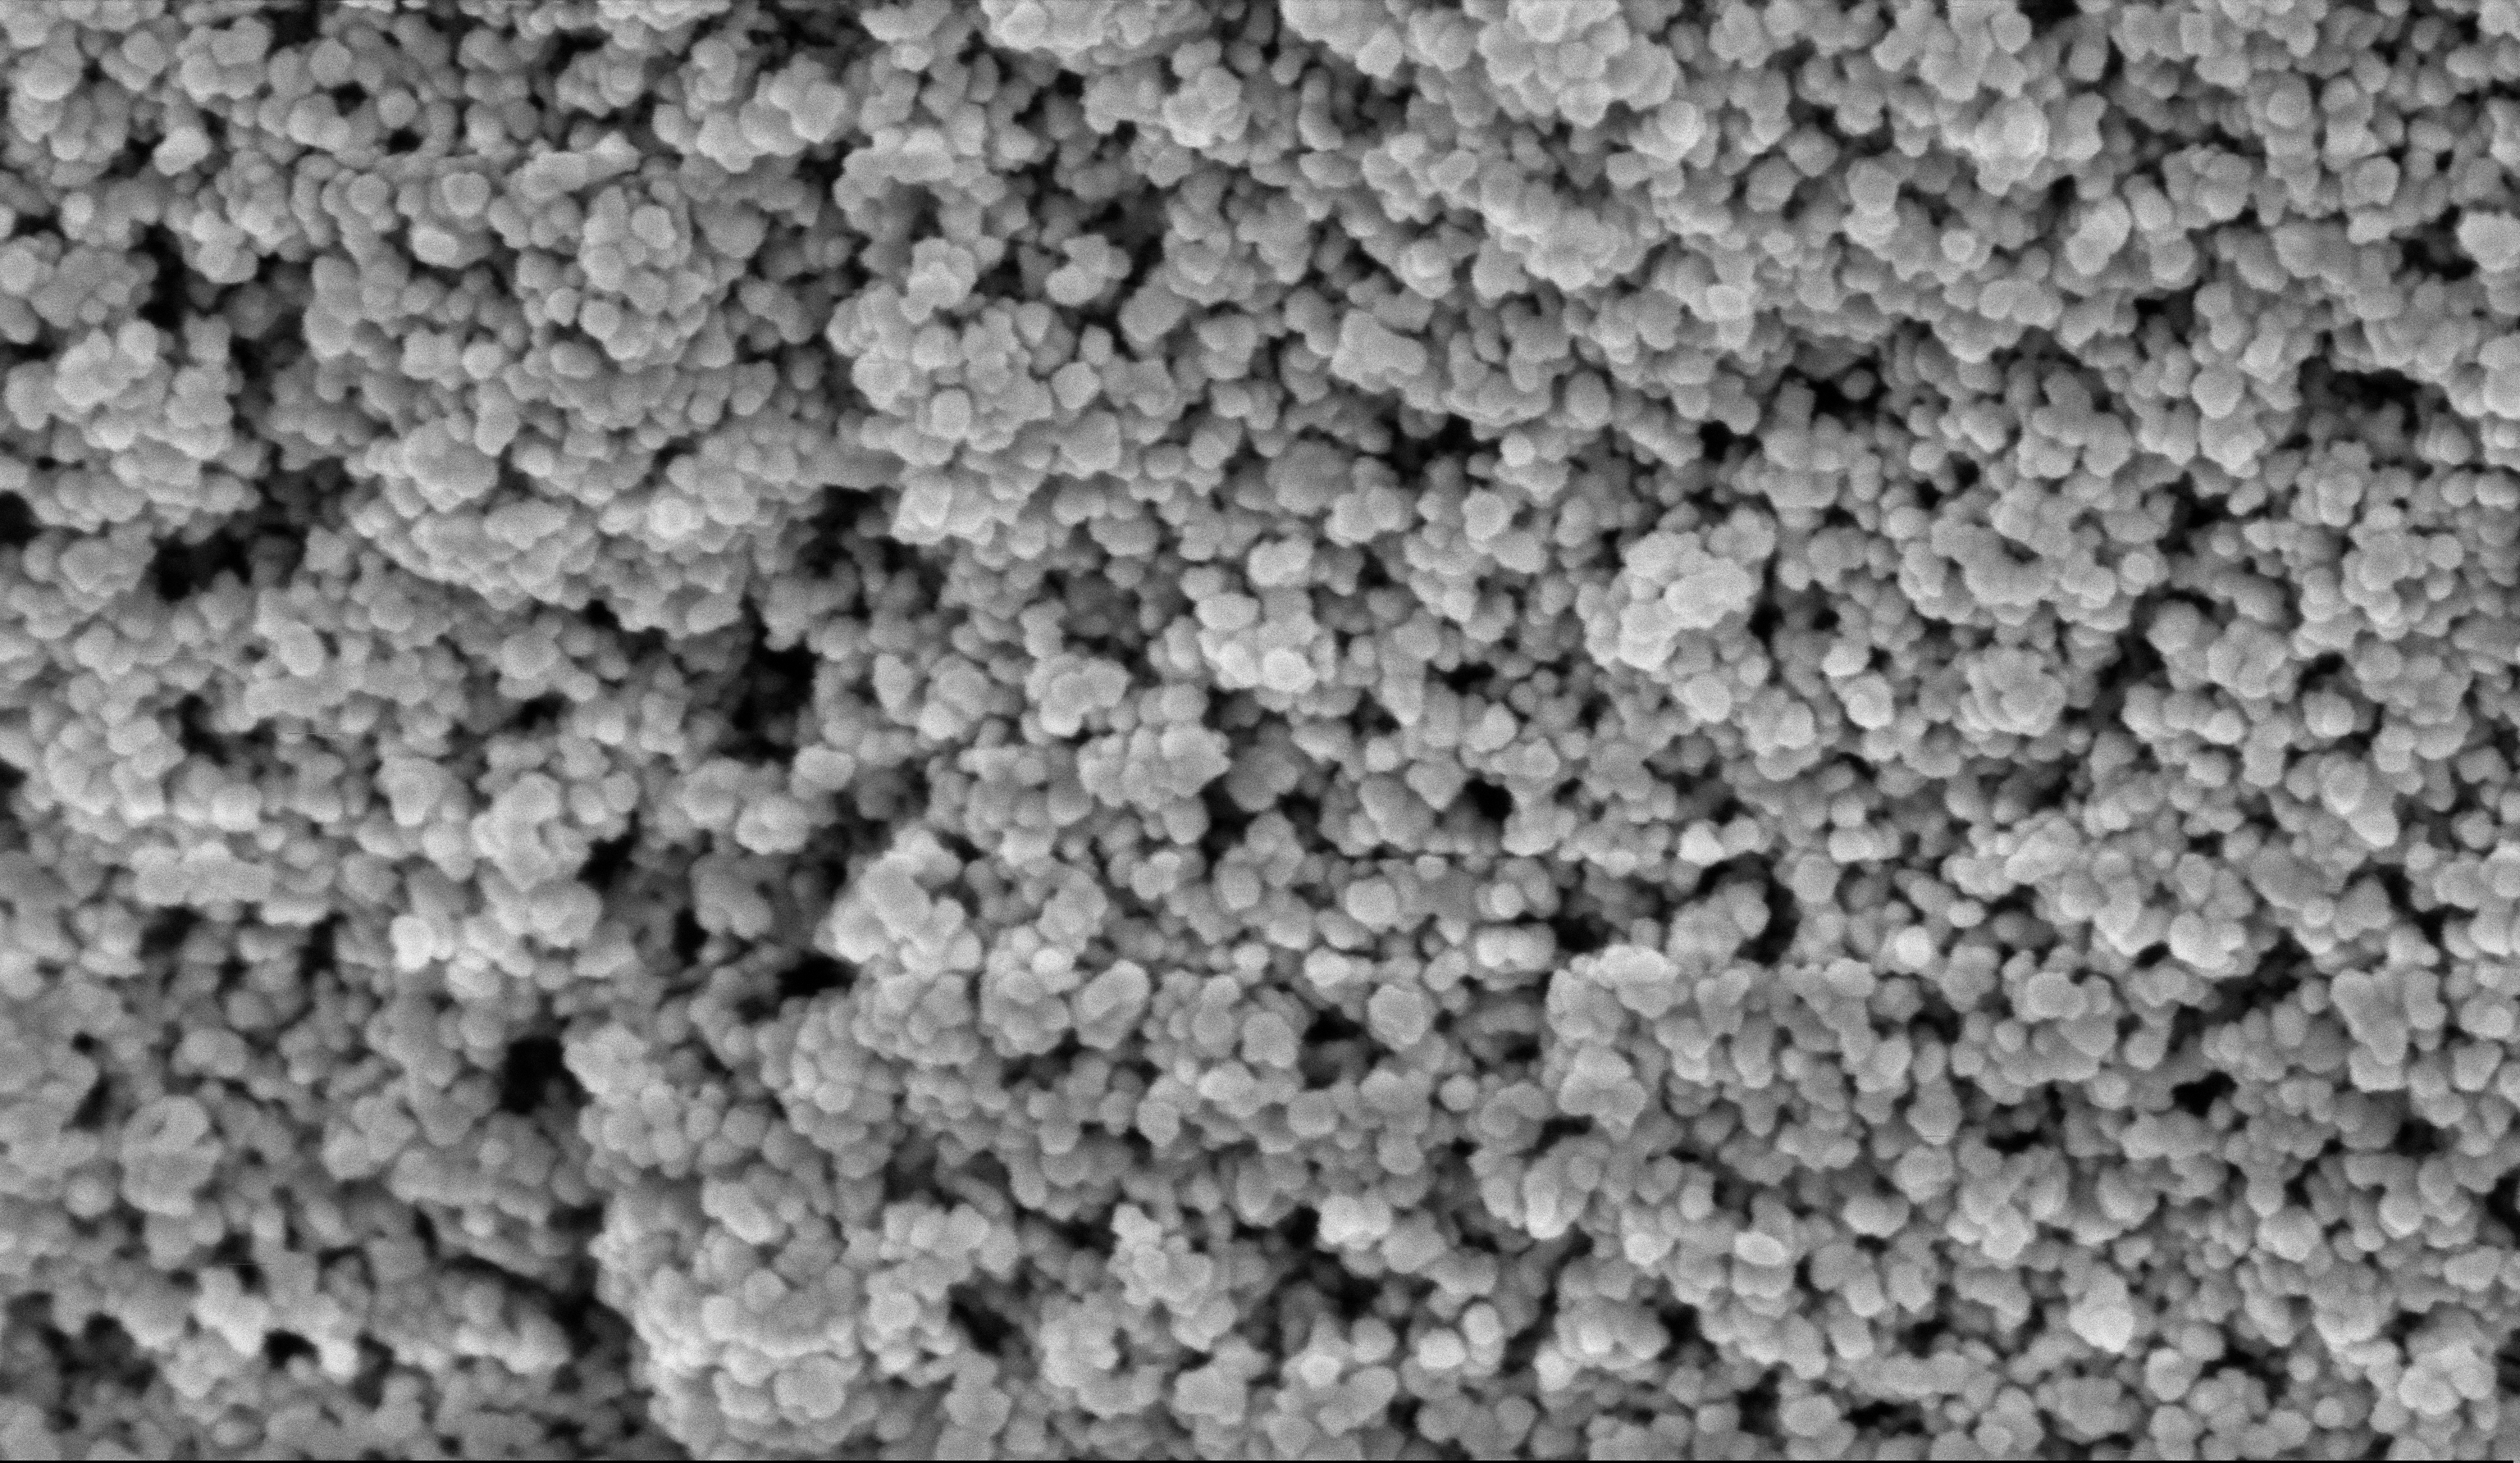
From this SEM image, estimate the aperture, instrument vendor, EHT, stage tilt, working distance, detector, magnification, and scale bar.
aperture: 30 µm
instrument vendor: Zeiss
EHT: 5 kV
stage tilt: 0°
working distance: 6 mm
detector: InLens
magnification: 135 K X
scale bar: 100 nm